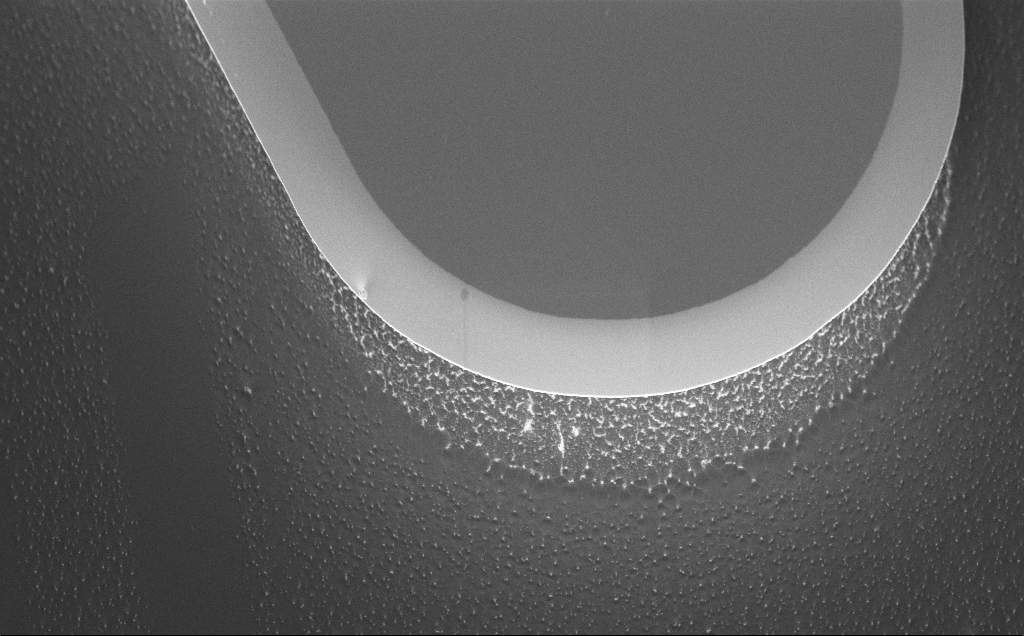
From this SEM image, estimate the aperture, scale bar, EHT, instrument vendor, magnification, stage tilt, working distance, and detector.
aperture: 30 µm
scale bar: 20000 nm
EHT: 5 kV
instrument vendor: Zeiss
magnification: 3.26 K X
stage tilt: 45°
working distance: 8 mm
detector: InLens